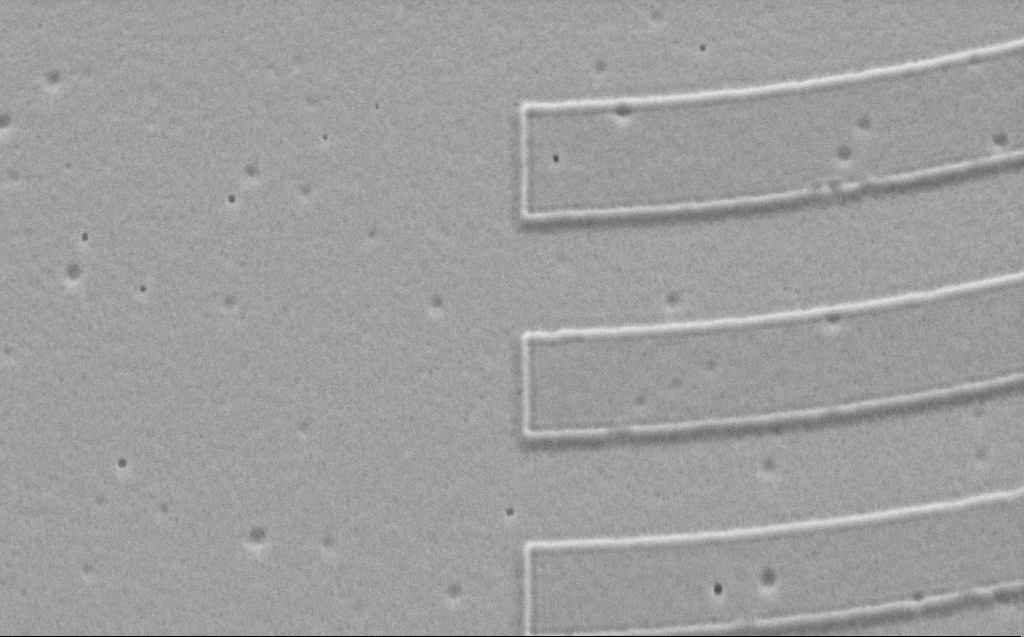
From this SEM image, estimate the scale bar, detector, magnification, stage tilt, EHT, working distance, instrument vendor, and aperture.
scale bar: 1000 nm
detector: SE2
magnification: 34.95 K X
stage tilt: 41.9°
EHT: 3 kV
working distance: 9 mm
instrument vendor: Zeiss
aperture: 30 µm